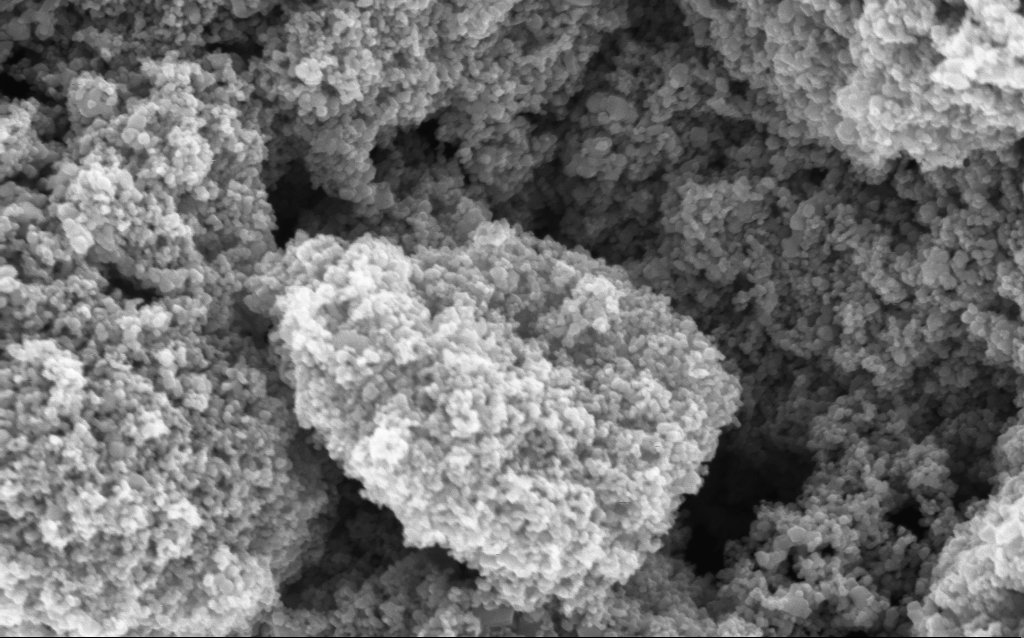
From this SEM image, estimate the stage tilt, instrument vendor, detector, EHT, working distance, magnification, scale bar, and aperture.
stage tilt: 0°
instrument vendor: Zeiss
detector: InLens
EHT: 5 kV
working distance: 4.7 mm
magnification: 114.65 K X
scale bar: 200 nm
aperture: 30 µm